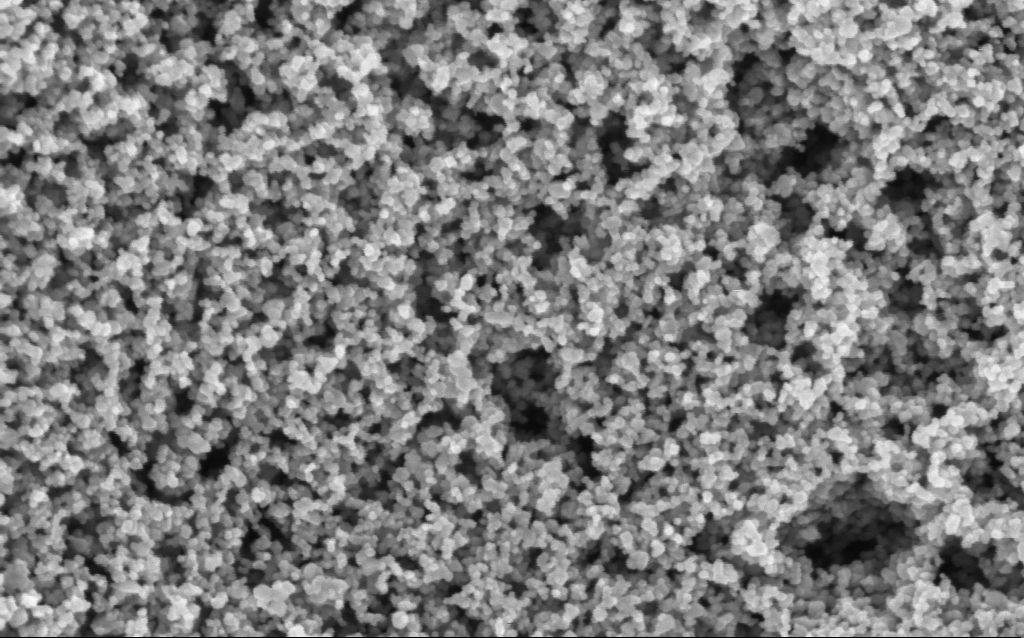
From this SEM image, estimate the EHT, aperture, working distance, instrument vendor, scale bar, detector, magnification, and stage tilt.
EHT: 3 kV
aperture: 30 µm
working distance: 7.6 mm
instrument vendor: Zeiss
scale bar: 100 nm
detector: InLens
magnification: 130 K X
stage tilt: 0°